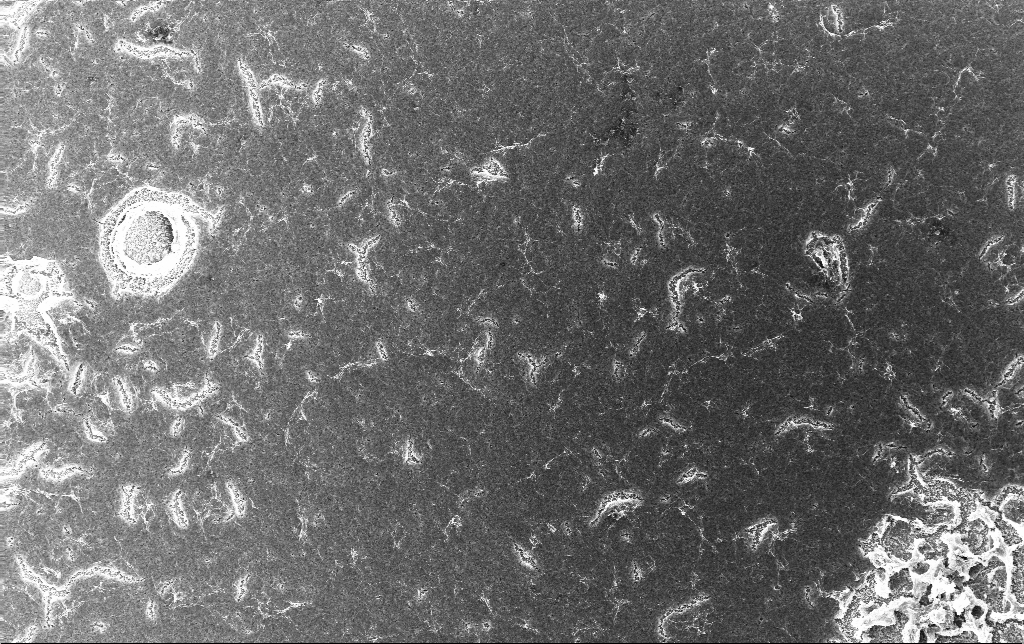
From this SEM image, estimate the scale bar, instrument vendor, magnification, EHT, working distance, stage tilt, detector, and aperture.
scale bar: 20000 nm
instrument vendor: Zeiss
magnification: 1 K X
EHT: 10 kV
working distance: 3 mm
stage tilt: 0°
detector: InLens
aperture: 30 µm